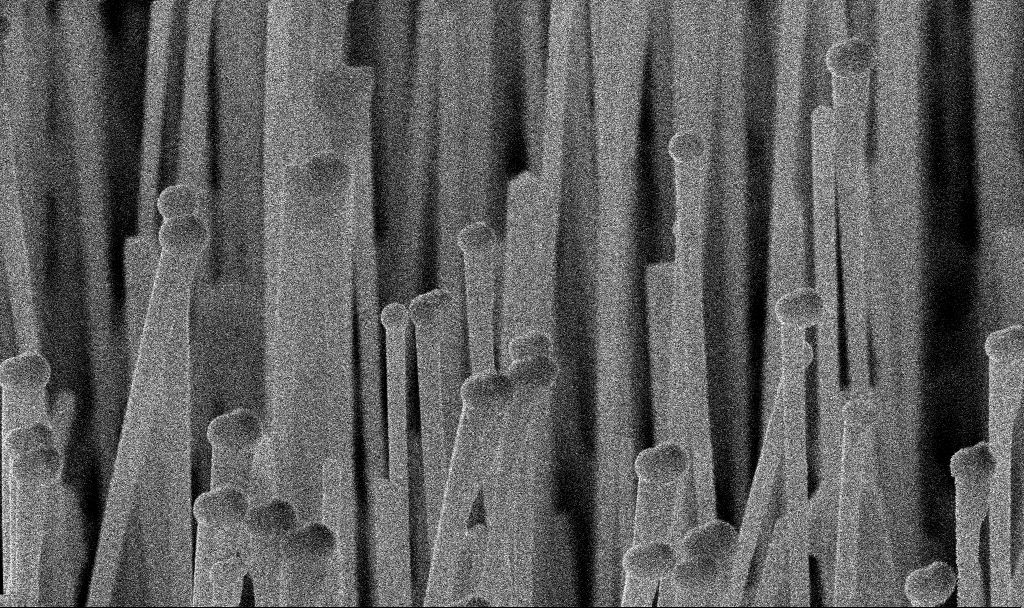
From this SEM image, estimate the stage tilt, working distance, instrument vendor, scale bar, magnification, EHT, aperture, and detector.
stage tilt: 45°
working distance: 7.1 mm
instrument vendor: Zeiss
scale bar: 1000 nm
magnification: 71.94 K X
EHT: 10 kV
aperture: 30 µm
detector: InLens